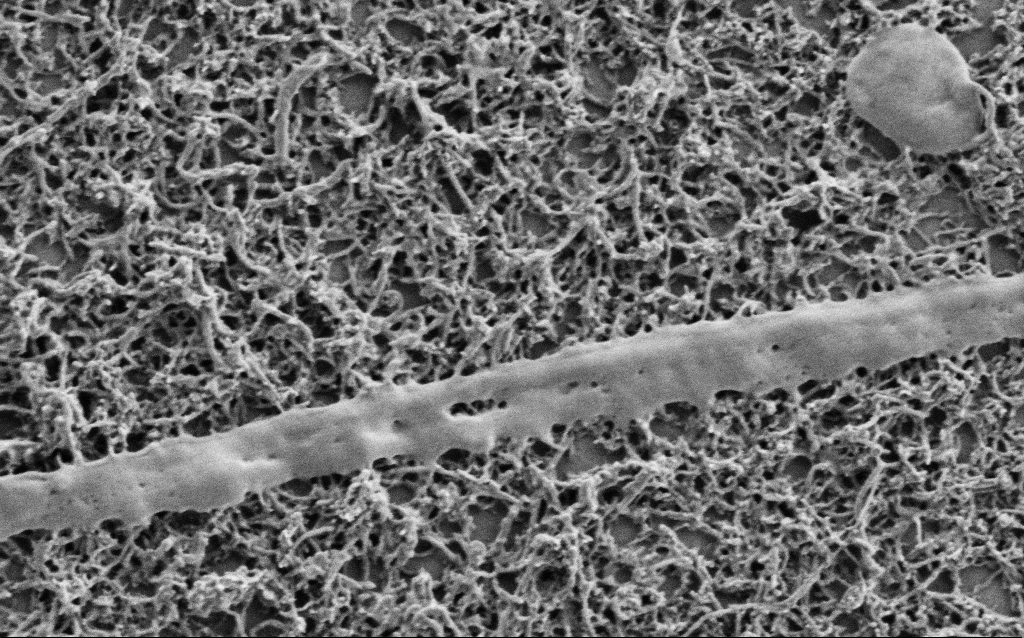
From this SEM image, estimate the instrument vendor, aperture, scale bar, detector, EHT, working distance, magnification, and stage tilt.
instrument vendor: Zeiss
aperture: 30 µm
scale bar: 1000 nm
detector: SE2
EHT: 1 kV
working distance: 7 mm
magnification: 50 K X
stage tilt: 0°